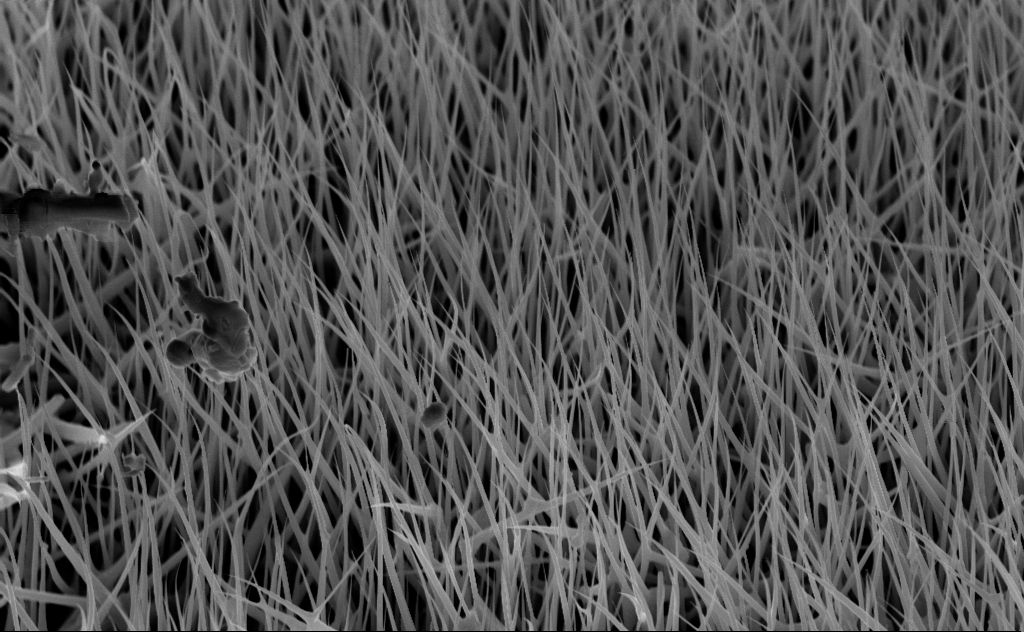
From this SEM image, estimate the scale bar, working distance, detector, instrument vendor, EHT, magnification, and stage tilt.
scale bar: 2000 nm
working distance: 6 mm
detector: InLens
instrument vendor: Zeiss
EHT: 10 kV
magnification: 20 K X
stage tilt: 45°